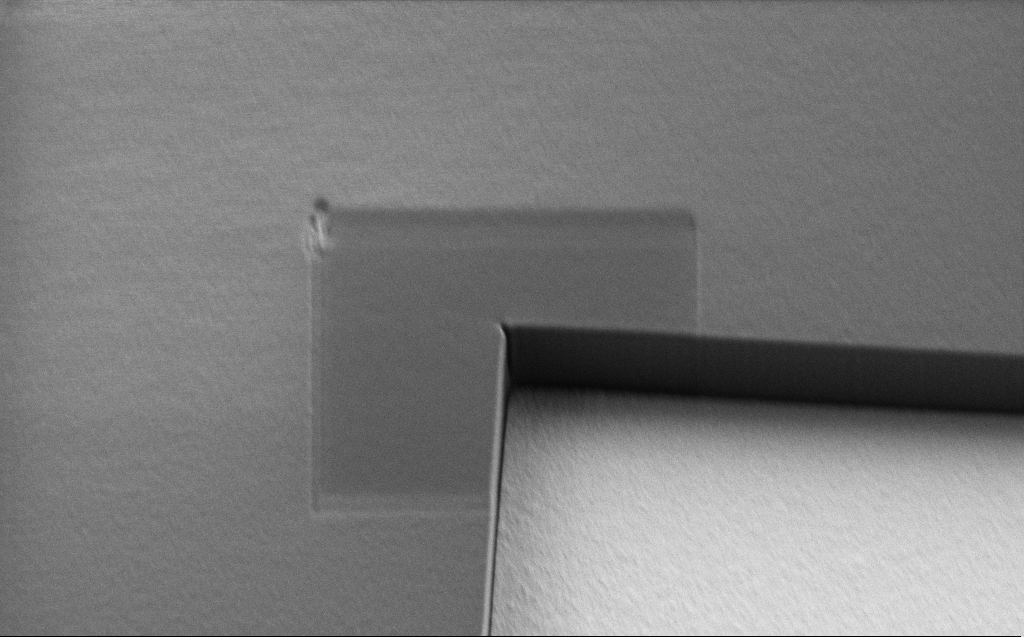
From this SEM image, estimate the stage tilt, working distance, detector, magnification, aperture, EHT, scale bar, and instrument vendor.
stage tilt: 30°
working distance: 6 mm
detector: SE2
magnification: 17.68 K X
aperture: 30 µm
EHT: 1.1 kV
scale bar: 2000 nm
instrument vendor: Zeiss